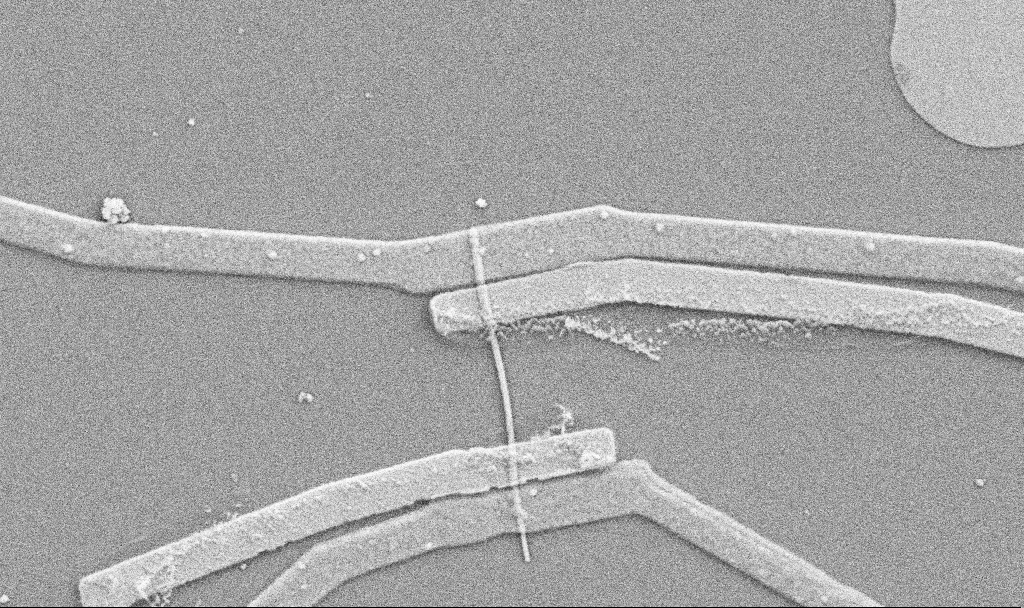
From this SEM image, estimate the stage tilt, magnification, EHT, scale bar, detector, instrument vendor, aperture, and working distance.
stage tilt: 0°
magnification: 20 K X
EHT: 5 kV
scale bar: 1000 nm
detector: SE2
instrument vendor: Zeiss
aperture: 30 µm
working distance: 10.7 mm